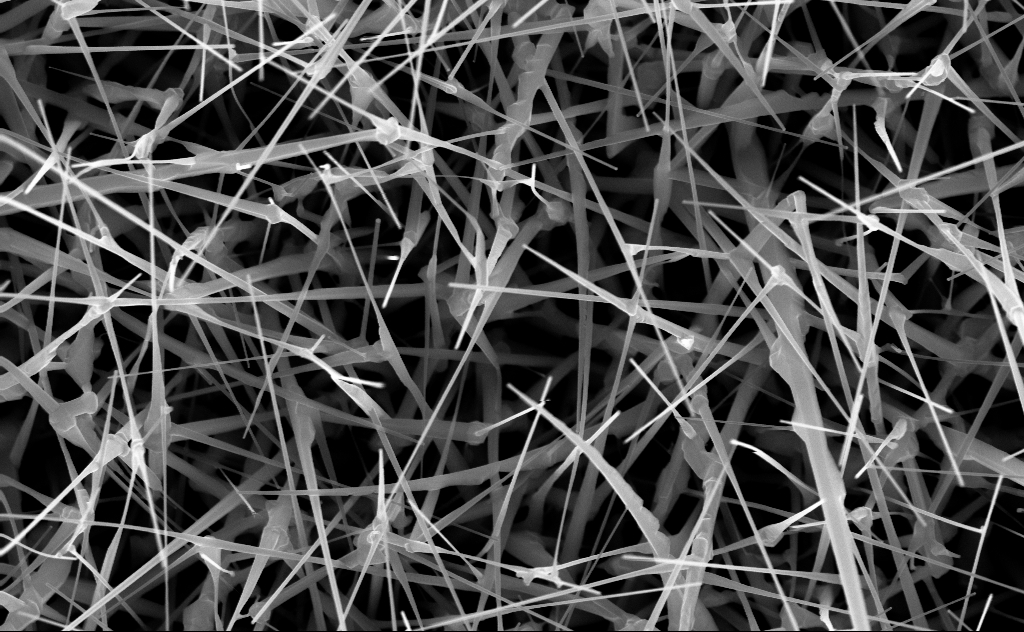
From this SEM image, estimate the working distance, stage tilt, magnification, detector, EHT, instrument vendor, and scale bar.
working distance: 6 mm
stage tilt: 0°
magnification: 40 K X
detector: InLens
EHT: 10 kV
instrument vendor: Zeiss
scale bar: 1000 nm